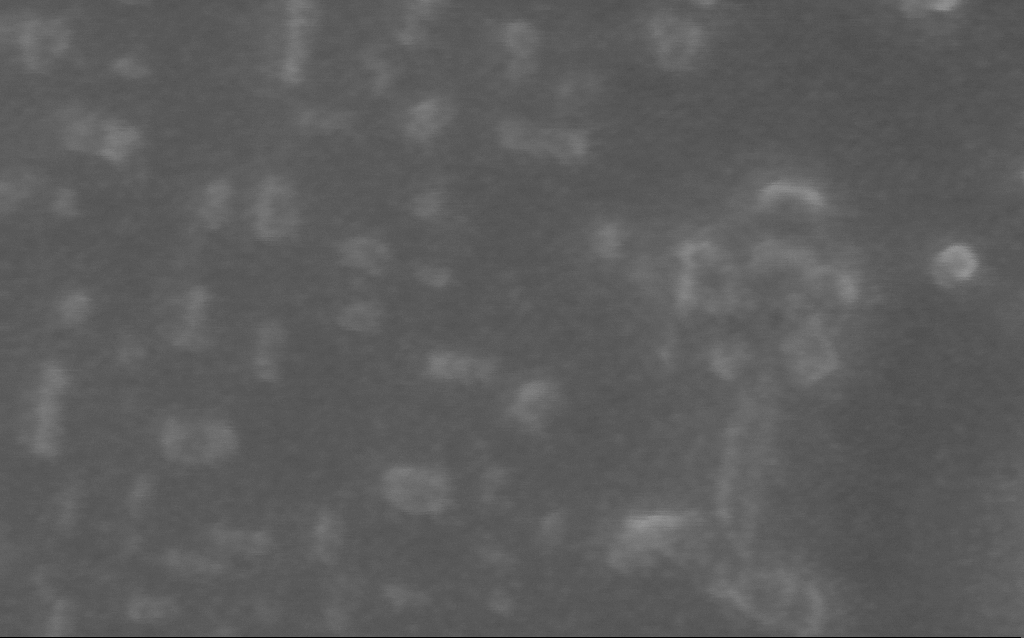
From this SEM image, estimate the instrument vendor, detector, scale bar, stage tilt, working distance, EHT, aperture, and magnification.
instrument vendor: Zeiss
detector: InLens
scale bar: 20 nm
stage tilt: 0°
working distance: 5 mm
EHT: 10 kV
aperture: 30 µm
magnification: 855.66 K X